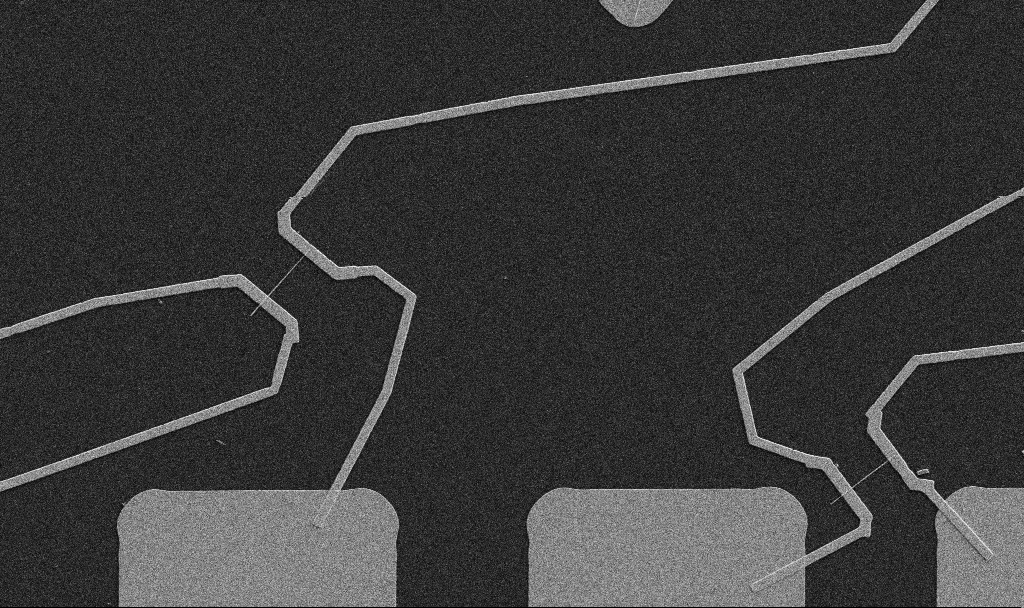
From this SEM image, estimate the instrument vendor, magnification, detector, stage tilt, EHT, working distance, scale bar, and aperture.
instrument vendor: Zeiss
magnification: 5 K X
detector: SE2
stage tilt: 0°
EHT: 5 kV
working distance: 10.7 mm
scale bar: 10000 nm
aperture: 30 µm